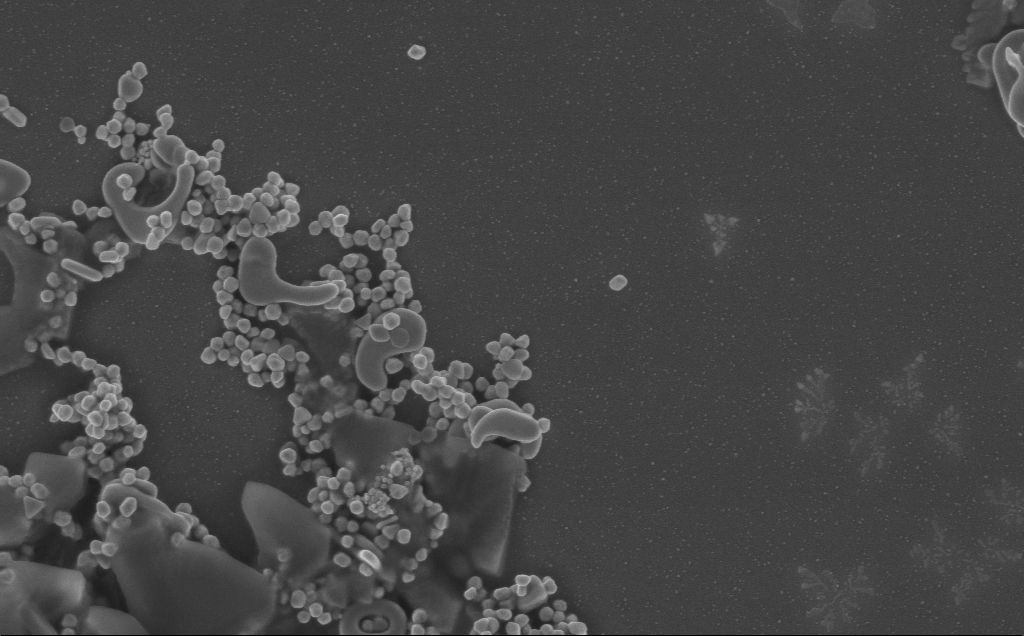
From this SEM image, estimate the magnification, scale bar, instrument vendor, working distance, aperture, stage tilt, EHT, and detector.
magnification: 40 K X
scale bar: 1000 nm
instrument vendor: Zeiss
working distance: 3 mm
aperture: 30 µm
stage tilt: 0°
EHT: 5 kV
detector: InLens